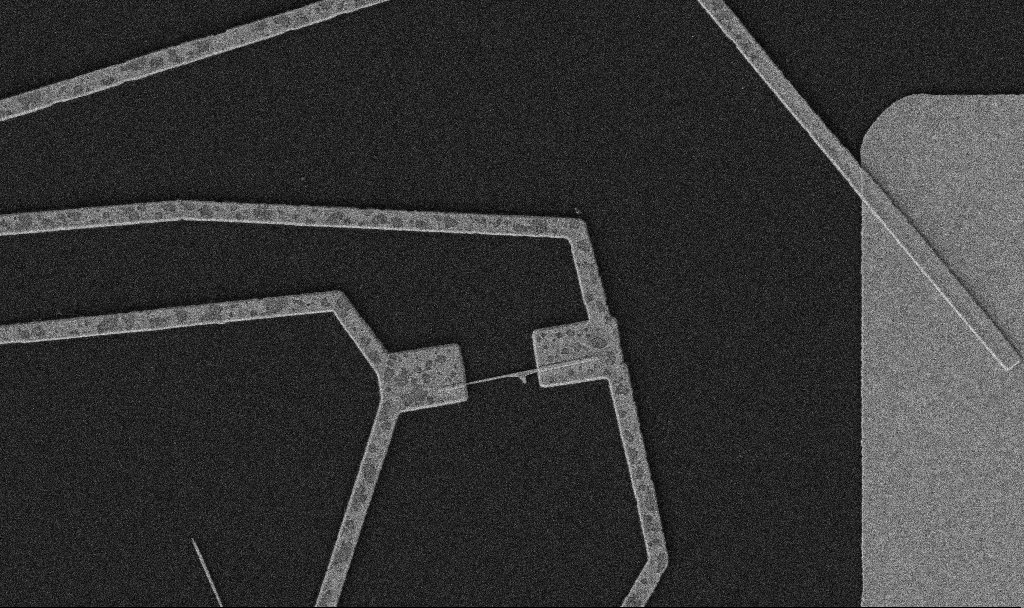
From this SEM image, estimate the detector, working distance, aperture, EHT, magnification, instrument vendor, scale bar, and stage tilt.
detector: SE2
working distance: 10.7 mm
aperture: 30 µm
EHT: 5 kV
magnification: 10 K X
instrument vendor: Zeiss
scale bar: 2000 nm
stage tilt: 0°